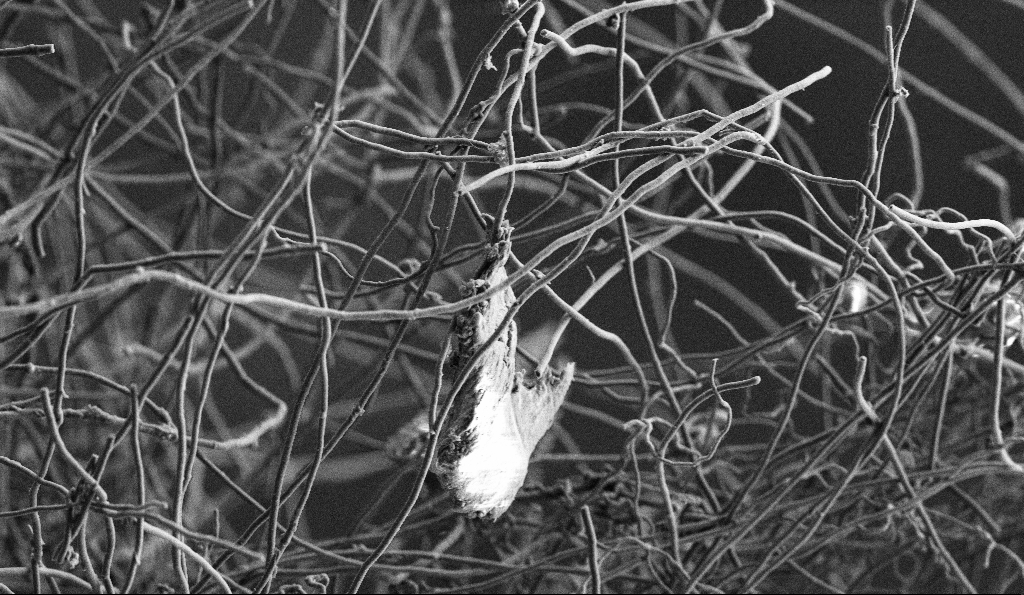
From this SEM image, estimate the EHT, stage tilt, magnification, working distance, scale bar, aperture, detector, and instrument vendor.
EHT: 3 kV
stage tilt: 0°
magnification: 5 K X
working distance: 5.9 mm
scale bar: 10000 nm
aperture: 30 µm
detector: SE2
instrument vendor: Zeiss